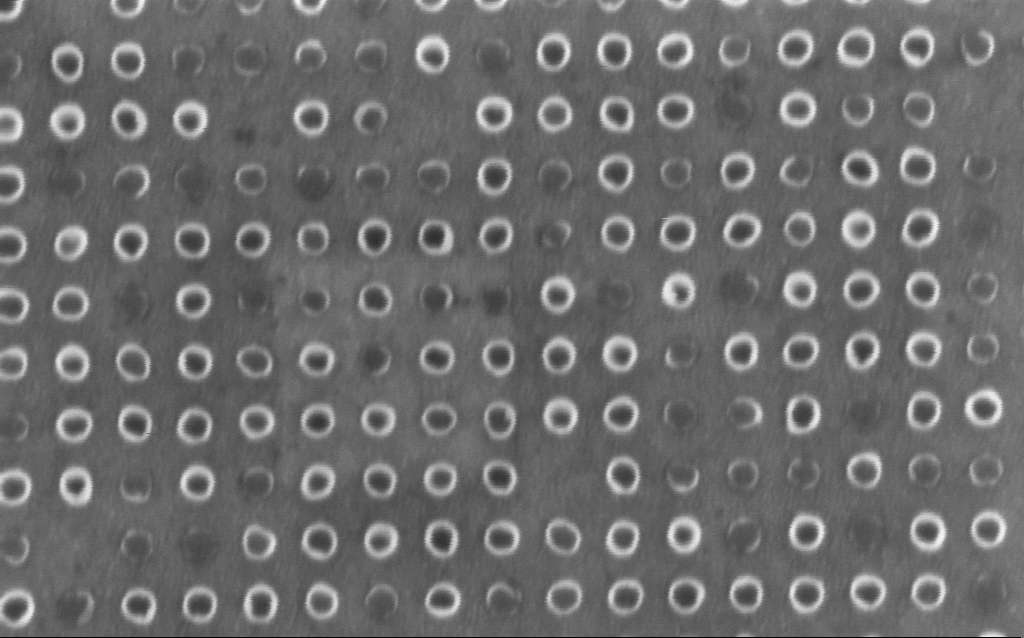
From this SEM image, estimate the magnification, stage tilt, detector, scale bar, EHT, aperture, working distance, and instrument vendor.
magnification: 158.02 K X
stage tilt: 0°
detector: InLens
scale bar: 200 nm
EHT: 1.5 kV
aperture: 30 µm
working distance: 5.9 mm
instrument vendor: Zeiss